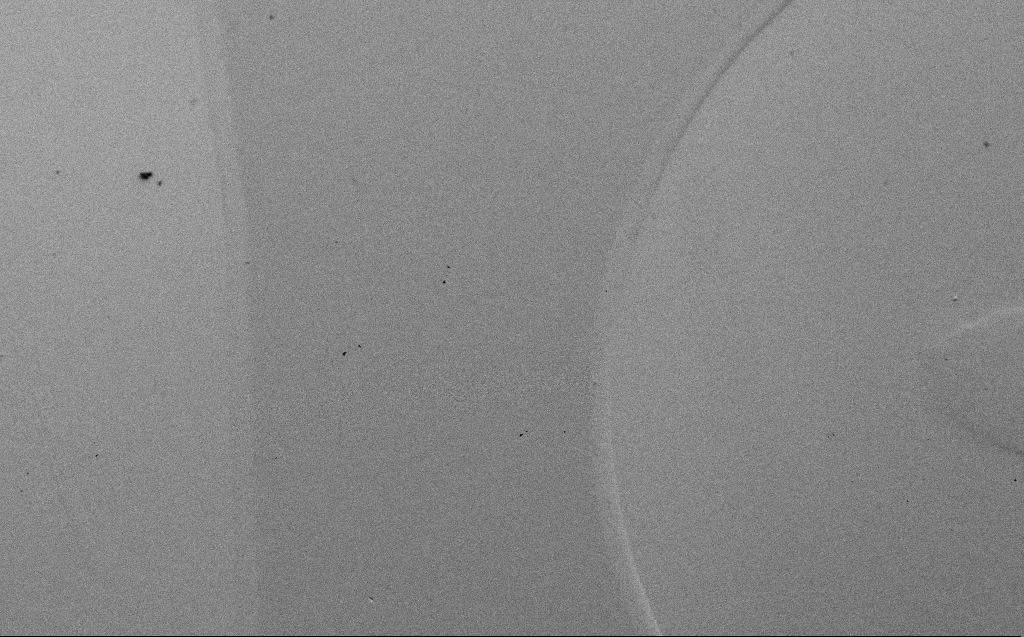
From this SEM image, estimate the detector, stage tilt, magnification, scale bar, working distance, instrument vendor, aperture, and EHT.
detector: SE2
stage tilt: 45°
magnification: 0.202 K X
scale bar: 100000 nm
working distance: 8 mm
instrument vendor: Zeiss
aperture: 30 µm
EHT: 5 kV